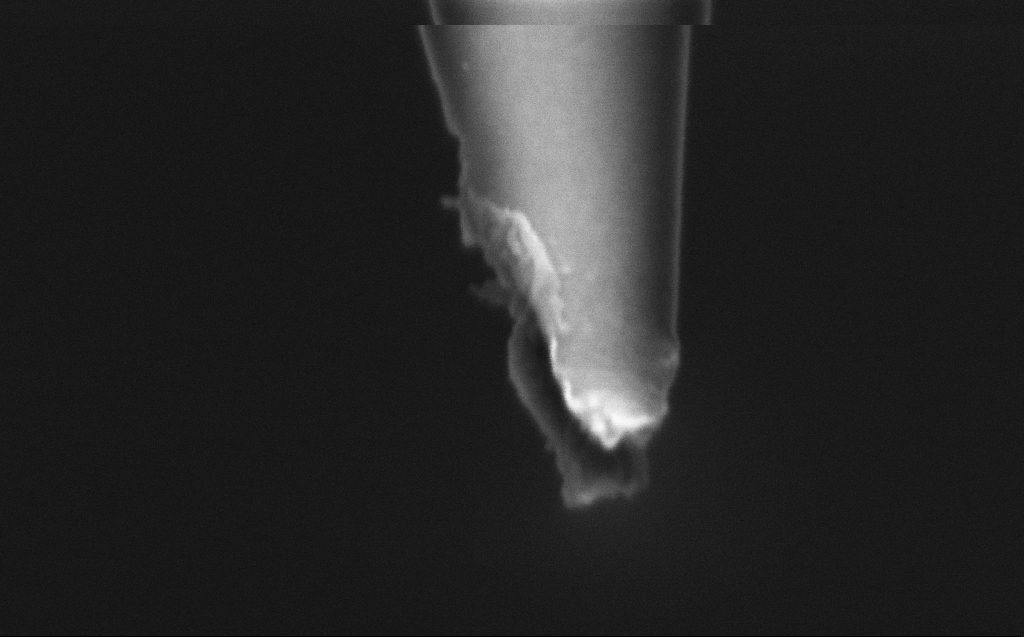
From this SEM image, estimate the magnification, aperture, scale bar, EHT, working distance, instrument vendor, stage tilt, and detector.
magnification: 250 K X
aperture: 30 µm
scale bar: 200 nm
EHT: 2 kV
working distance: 6 mm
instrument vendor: Zeiss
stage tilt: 45°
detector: InLens